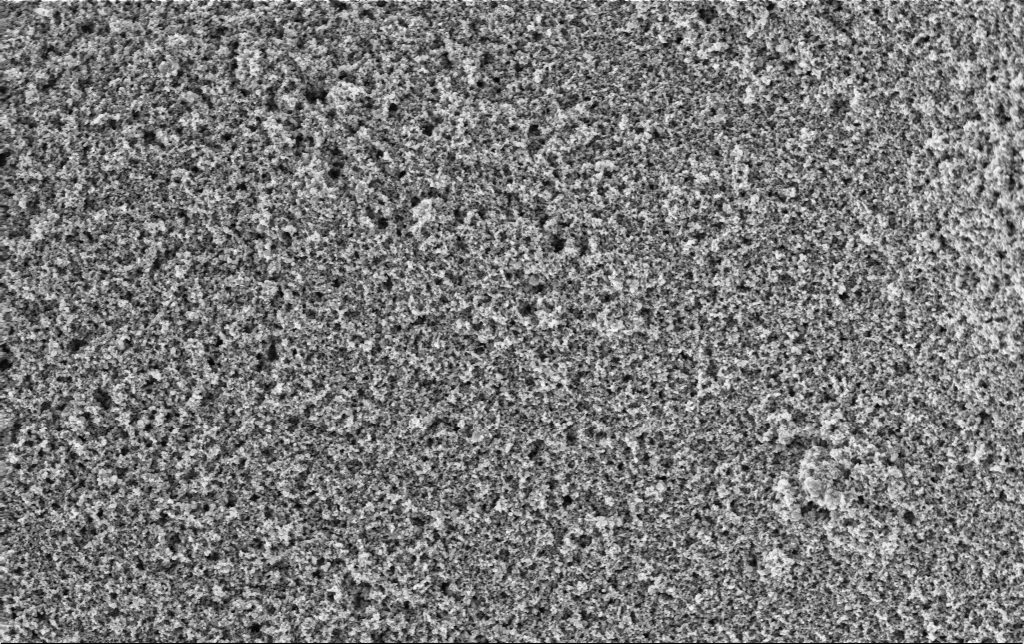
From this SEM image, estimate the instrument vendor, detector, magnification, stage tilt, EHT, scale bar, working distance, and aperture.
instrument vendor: Zeiss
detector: InLens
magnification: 30 K X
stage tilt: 0°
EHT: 3 kV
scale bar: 2000 nm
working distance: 2.8 mm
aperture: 30 µm